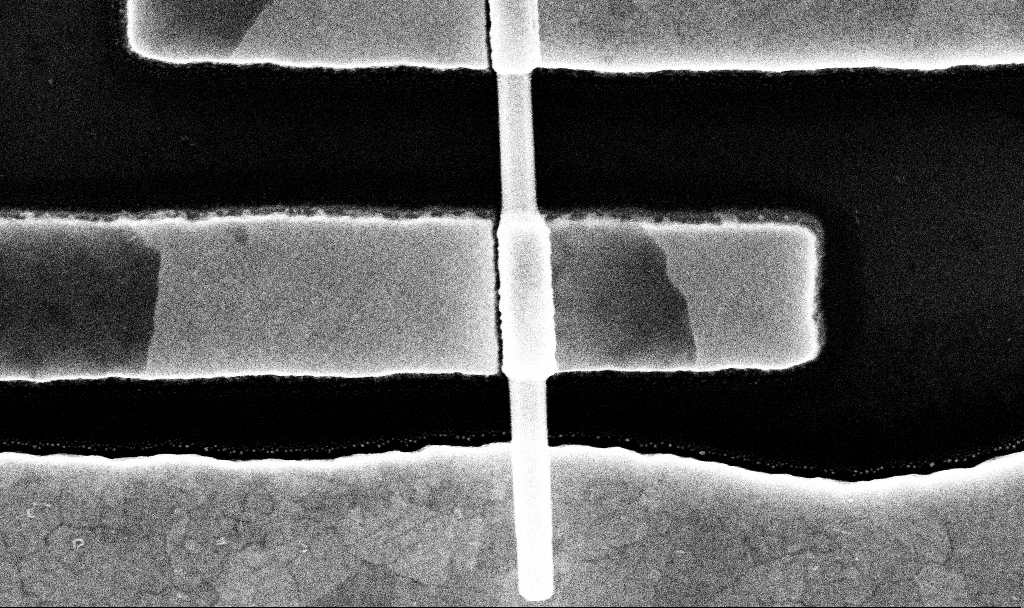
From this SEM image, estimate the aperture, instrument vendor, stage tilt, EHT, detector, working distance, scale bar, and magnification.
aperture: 30 µm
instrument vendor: Zeiss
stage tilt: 0°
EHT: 10 kV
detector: InLens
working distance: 6.8 mm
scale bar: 200 nm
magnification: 98.91 K X